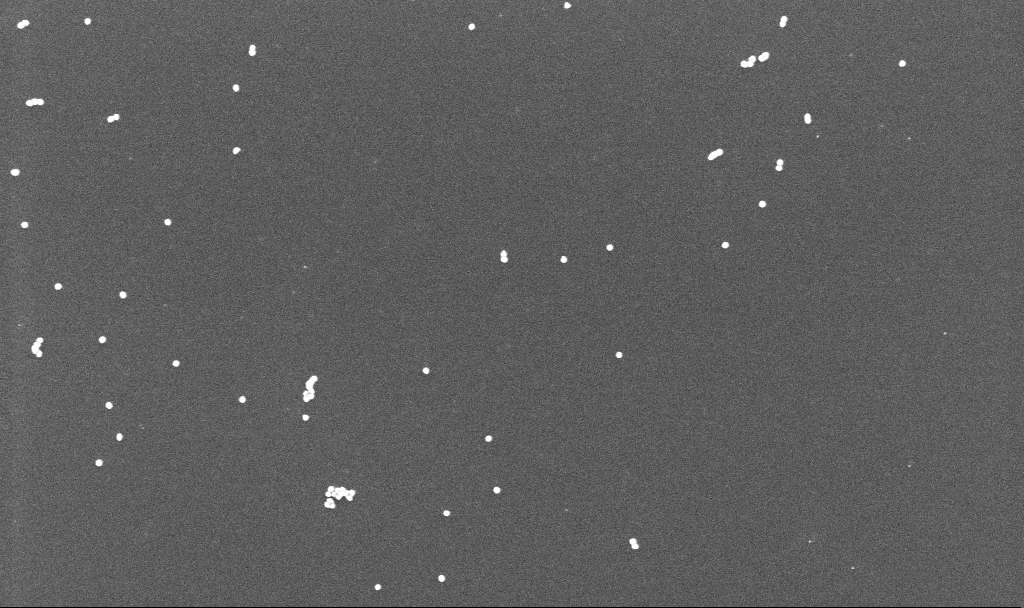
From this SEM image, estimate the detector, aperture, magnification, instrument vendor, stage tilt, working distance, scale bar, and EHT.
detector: InLens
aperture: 30 µm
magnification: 100 K X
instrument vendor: Zeiss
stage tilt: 0°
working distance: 3.4 mm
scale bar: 200 nm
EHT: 10 kV